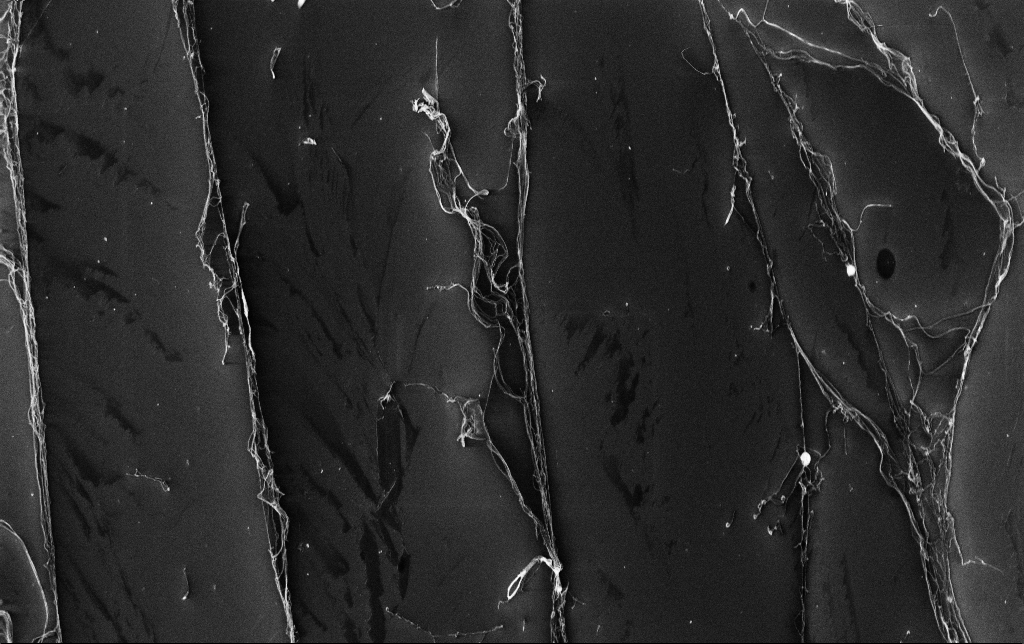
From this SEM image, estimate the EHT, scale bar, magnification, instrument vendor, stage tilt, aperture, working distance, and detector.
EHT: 10 kV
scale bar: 20000 nm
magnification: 1.31 K X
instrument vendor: Zeiss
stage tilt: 0°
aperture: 30 µm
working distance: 3.4 mm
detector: InLens